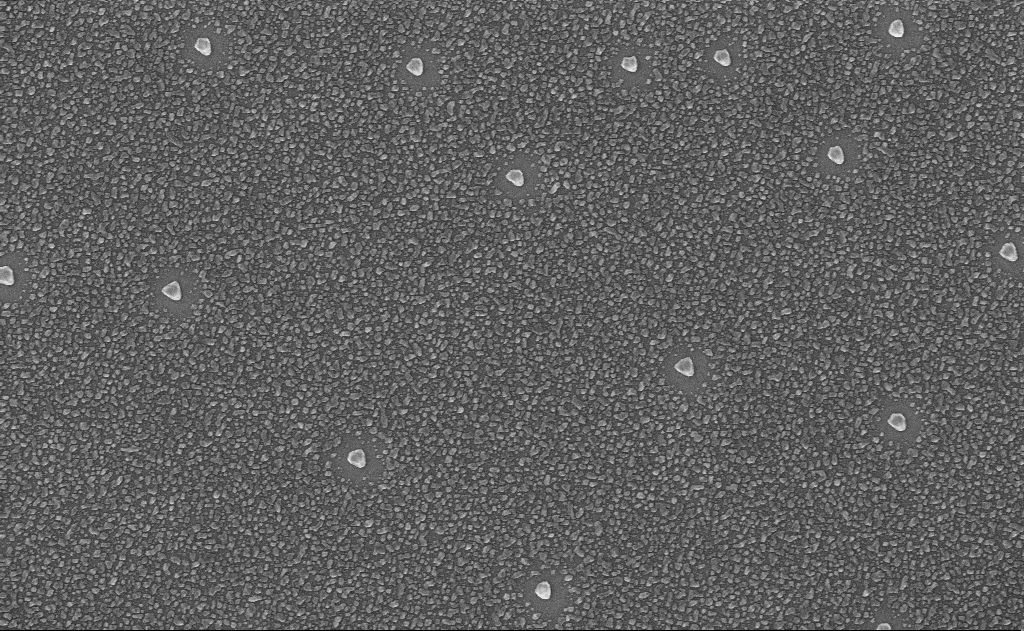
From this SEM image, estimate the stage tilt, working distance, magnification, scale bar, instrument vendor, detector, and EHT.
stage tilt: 0°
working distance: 15 mm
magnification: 40 K X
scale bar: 1000 nm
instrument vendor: Zeiss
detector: InLens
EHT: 10 kV